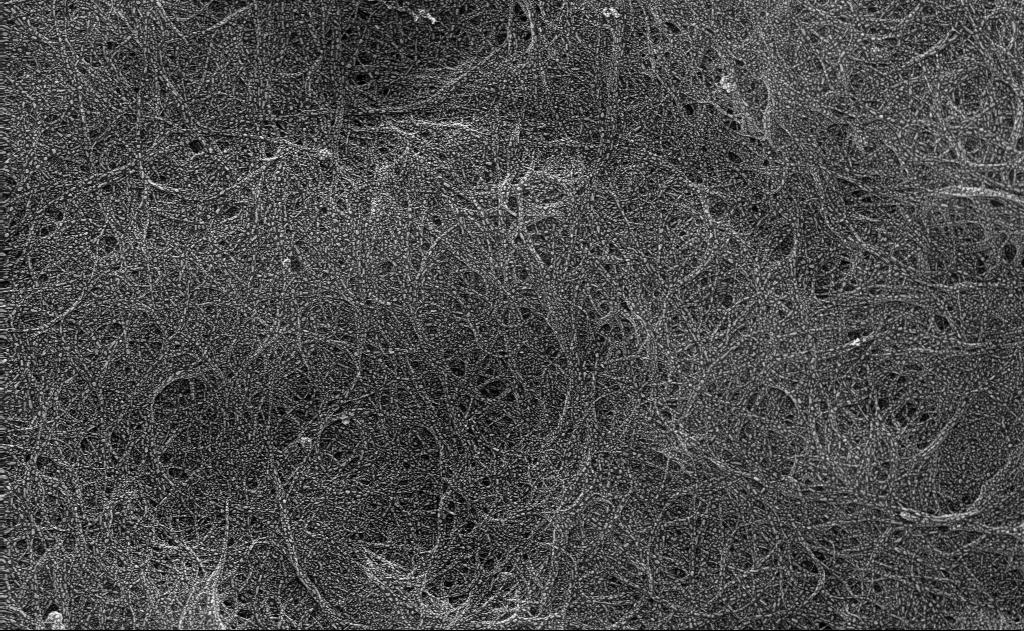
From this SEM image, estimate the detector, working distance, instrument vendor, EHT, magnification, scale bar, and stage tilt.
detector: InLens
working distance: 3 mm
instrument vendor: Zeiss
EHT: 10 kV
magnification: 36.1 K X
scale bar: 1000 nm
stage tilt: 0°